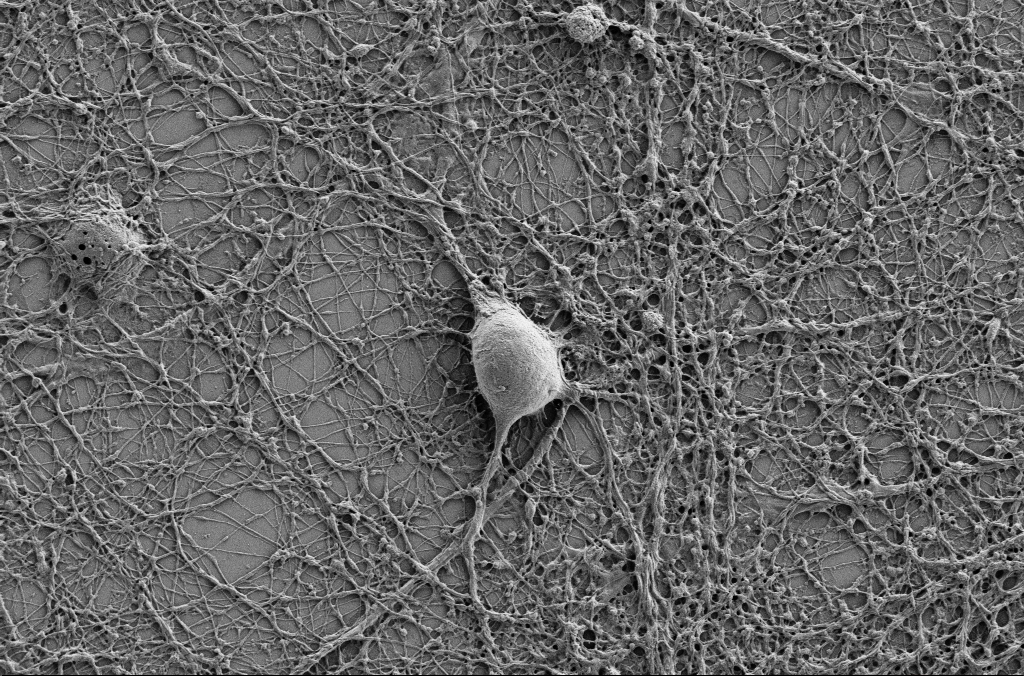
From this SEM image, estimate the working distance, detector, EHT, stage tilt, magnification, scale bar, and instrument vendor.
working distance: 4.1 mm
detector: SE2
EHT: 1 kV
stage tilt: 0°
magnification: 5 K X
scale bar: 10000 nm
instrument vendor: Zeiss